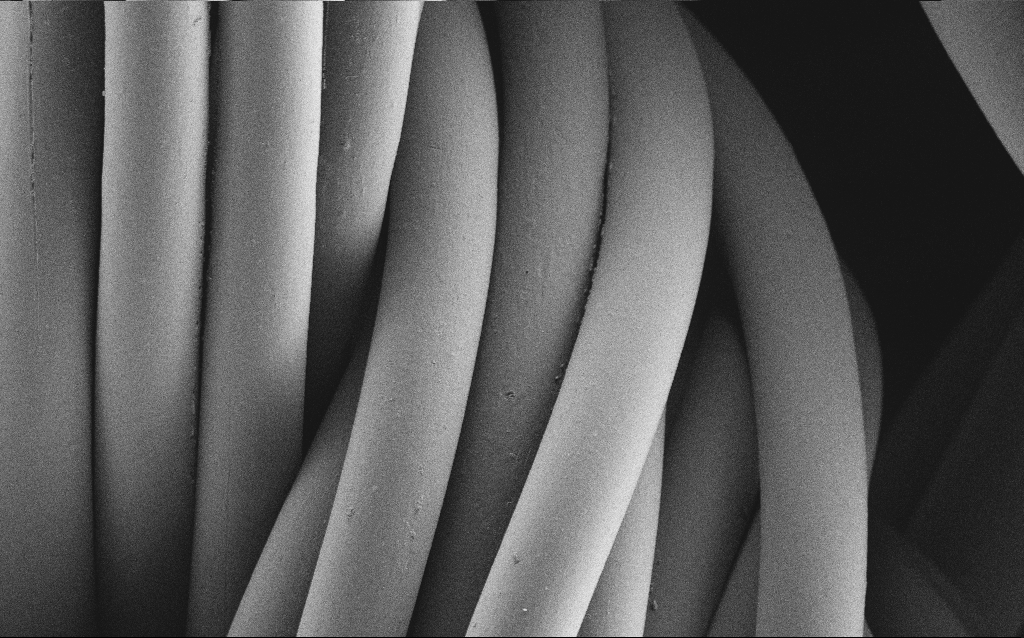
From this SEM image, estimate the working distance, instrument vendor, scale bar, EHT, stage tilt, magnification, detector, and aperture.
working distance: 4 mm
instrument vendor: Zeiss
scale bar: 20000 nm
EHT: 1 kV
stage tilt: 0°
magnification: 1.63 K X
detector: SE2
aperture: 30 µm